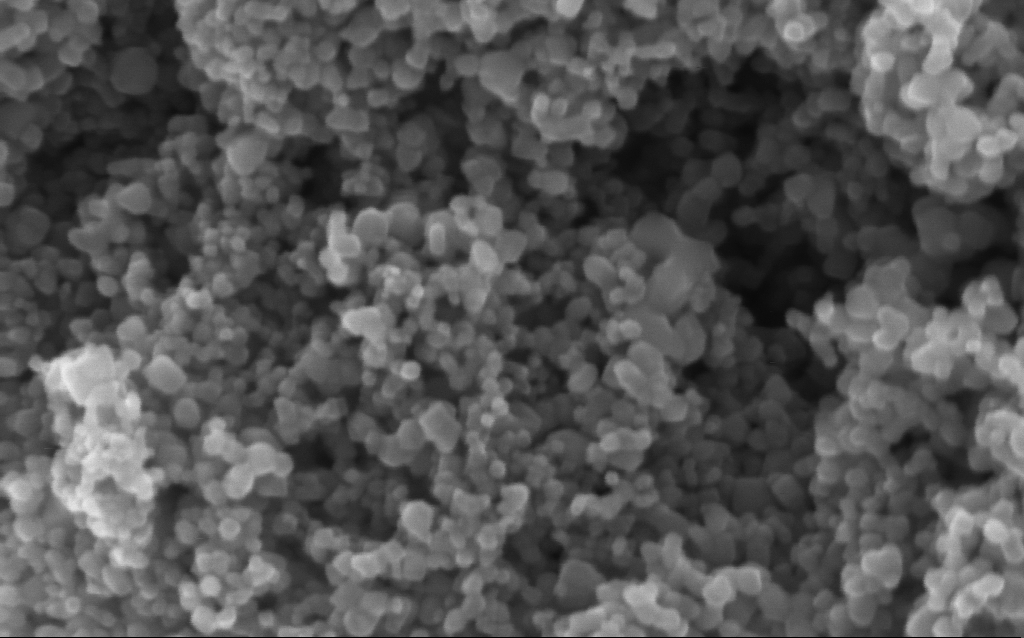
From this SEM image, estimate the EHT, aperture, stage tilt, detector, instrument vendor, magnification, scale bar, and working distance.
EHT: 5 kV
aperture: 30 µm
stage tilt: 0°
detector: InLens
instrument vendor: Zeiss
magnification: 294.05 K X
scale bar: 100 nm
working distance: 4.2 mm